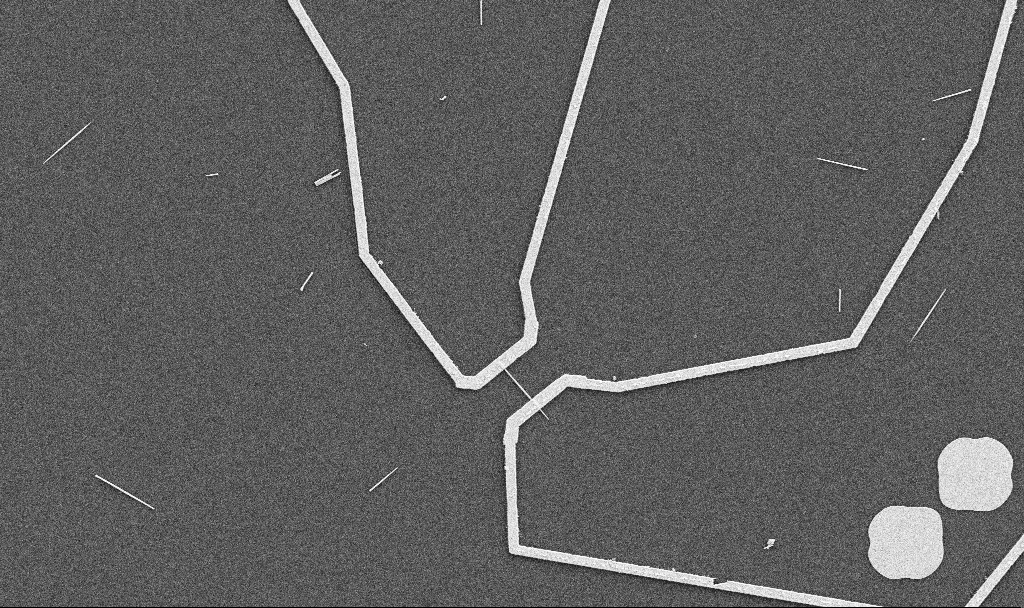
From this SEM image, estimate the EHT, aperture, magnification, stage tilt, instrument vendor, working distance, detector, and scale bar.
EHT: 5 kV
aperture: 30 µm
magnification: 5 K X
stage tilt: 0°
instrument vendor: Zeiss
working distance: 10.7 mm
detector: SE2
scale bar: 10000 nm